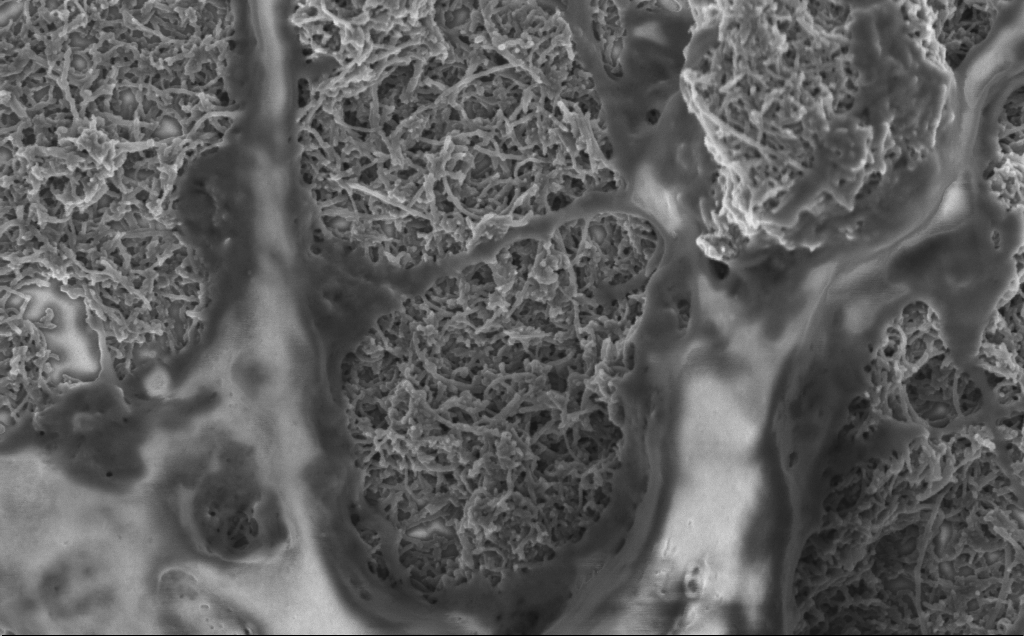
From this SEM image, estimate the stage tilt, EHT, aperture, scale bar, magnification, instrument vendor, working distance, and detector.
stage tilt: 0°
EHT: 2 kV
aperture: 30 µm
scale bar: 1000 nm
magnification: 50 K X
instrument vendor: Zeiss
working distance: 7.1 mm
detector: InLens